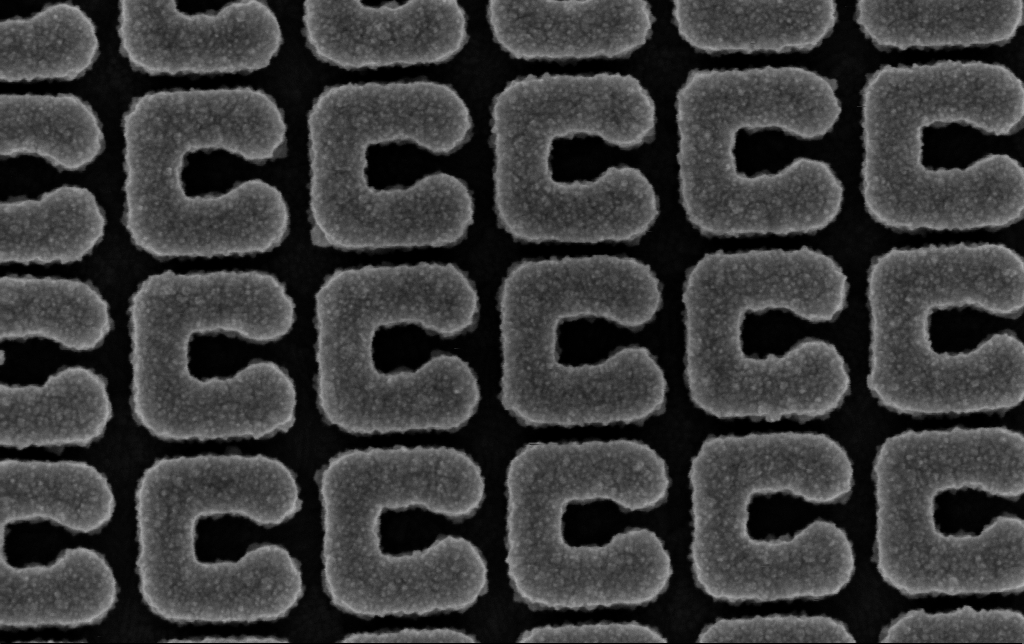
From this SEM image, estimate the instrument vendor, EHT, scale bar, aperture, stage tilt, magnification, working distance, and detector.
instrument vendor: Zeiss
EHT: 5 kV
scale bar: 200 nm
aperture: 30 µm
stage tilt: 0°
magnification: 147.98 K X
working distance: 4.9 mm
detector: InLens